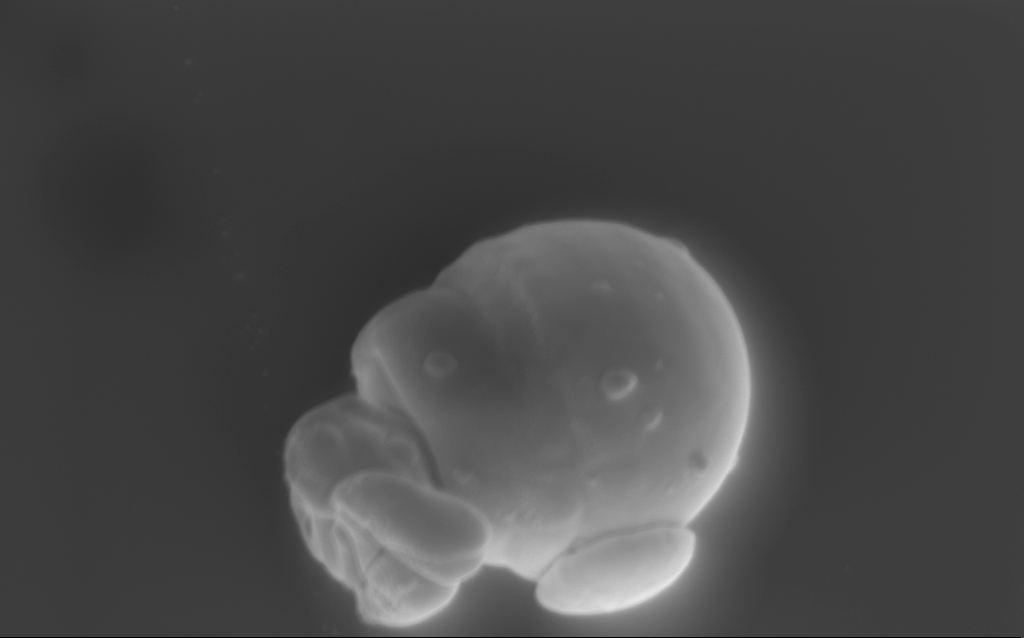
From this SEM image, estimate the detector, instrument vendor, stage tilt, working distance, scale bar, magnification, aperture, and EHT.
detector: InLens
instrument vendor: Zeiss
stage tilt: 22°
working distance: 6 mm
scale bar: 1000 nm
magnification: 52.23 K X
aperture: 120 µm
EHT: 4 kV